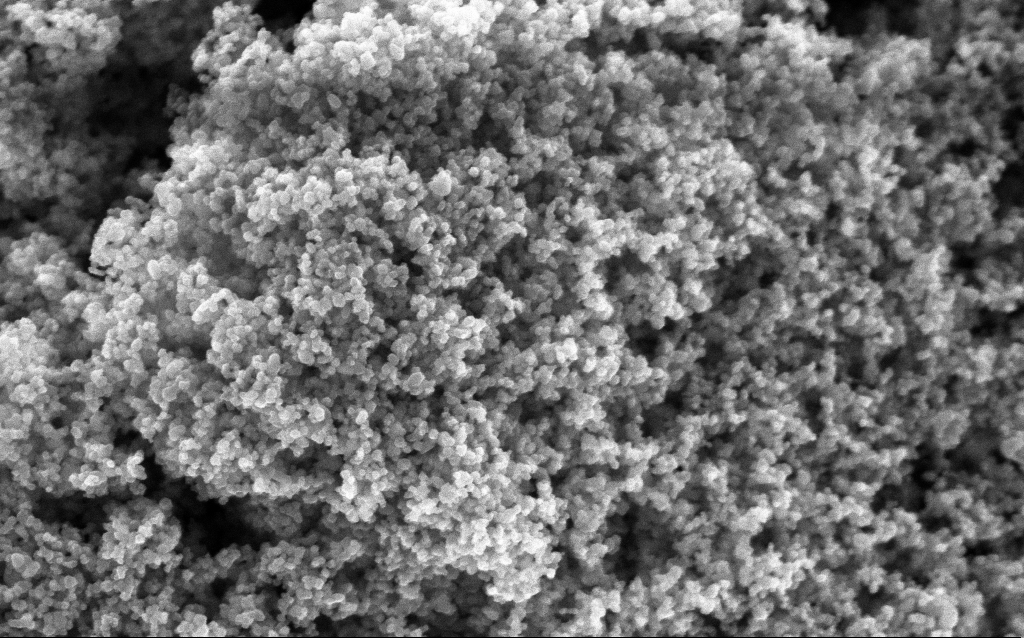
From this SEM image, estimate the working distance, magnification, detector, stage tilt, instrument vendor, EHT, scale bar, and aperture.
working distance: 2.9 mm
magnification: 130 K X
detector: InLens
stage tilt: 0°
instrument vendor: Zeiss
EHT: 5 kV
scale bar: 100 nm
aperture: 30 µm